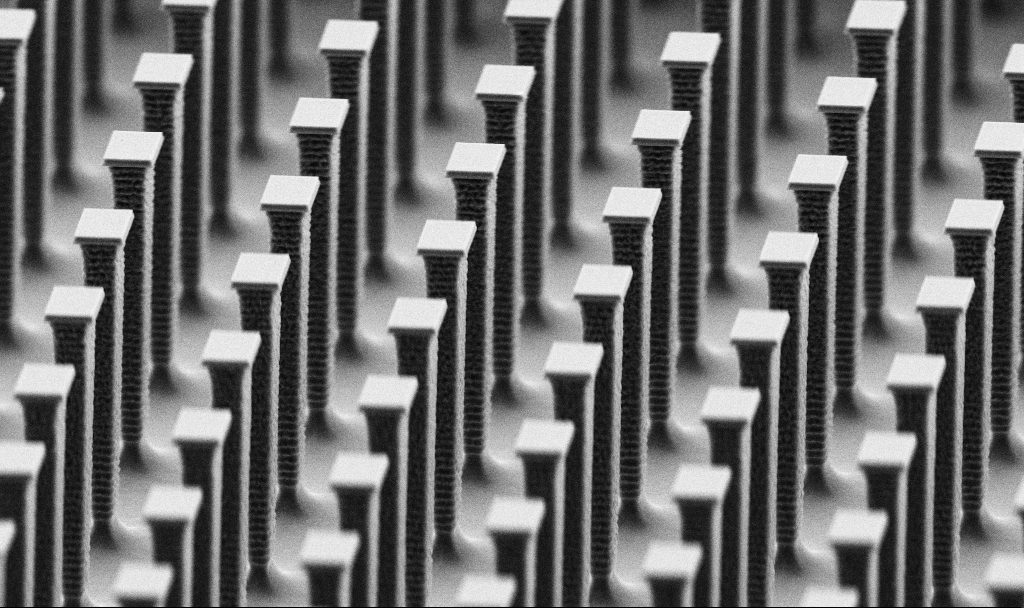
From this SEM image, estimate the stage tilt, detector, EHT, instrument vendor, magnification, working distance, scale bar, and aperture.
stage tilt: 70°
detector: SE2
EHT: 5 kV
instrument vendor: Zeiss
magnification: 6.54 K X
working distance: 5.6 mm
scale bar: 10000 nm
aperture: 30 µm